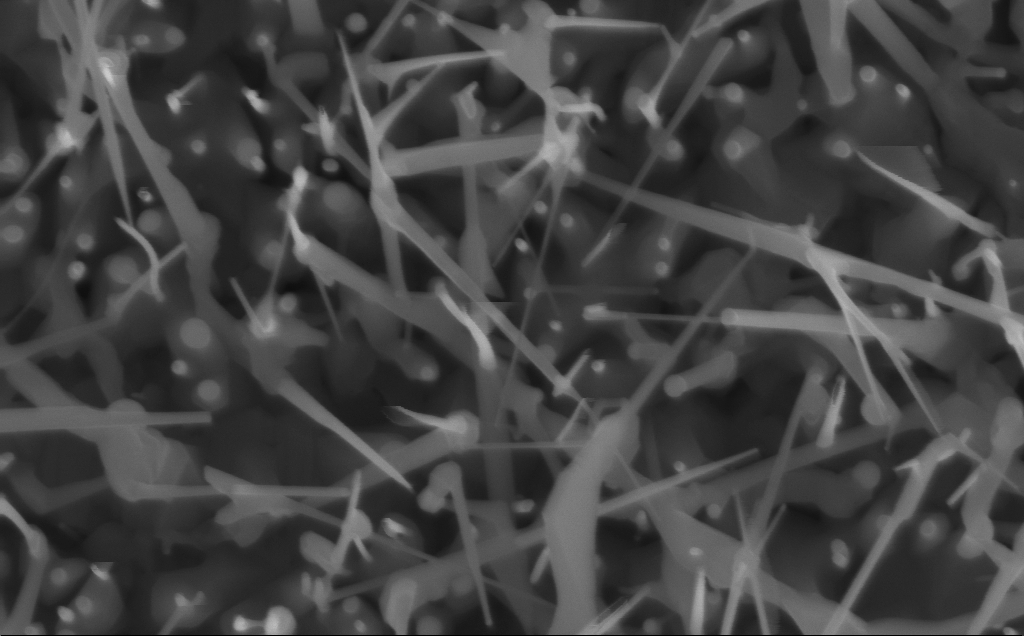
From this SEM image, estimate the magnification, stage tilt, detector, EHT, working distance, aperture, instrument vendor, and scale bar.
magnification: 84.85 K X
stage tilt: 0°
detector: InLens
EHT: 10 kV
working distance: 3 mm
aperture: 30 µm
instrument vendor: Zeiss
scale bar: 200 nm